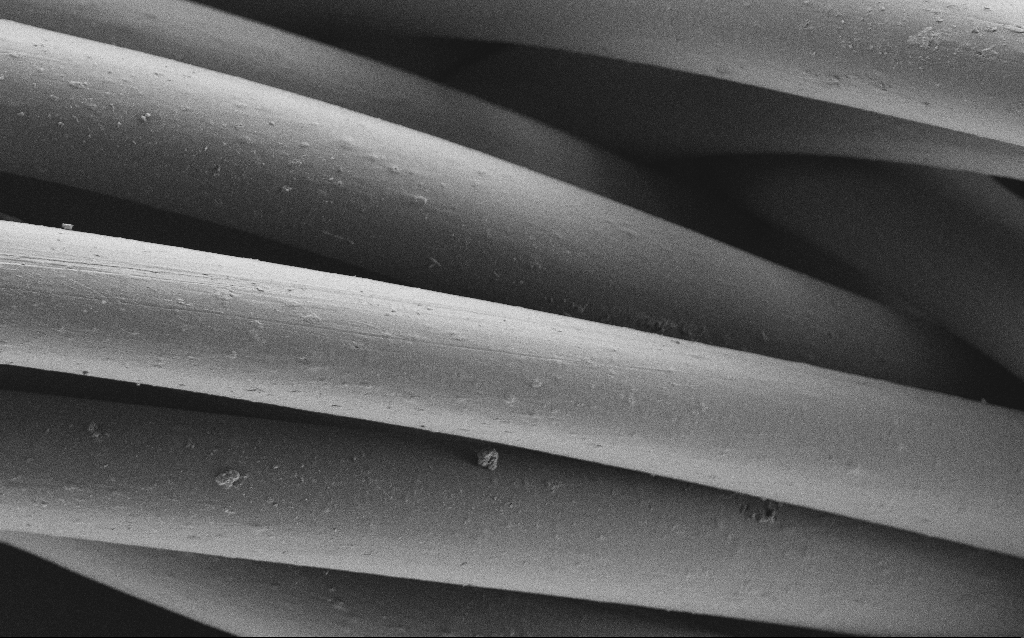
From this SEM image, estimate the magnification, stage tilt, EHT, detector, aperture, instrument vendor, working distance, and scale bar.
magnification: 2.28 K X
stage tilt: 0°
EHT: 1 kV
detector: SE2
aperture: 30 µm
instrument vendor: Zeiss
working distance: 4 mm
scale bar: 20000 nm